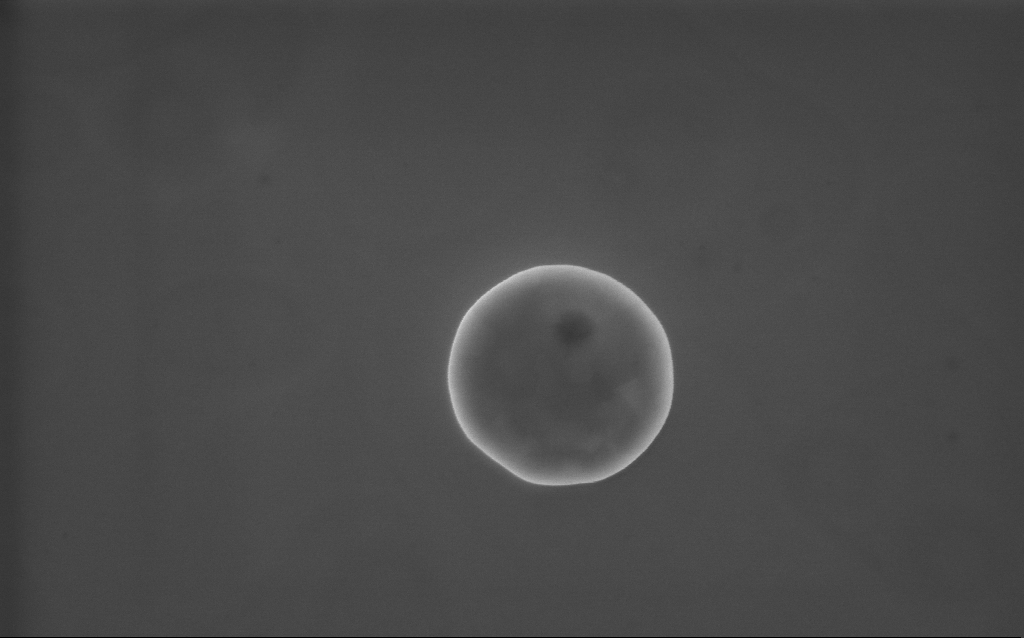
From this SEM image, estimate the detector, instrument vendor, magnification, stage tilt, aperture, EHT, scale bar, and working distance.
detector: InLens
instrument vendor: Zeiss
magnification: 65 K X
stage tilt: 0°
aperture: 30 µm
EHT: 10 kV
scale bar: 1000 nm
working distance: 3 mm